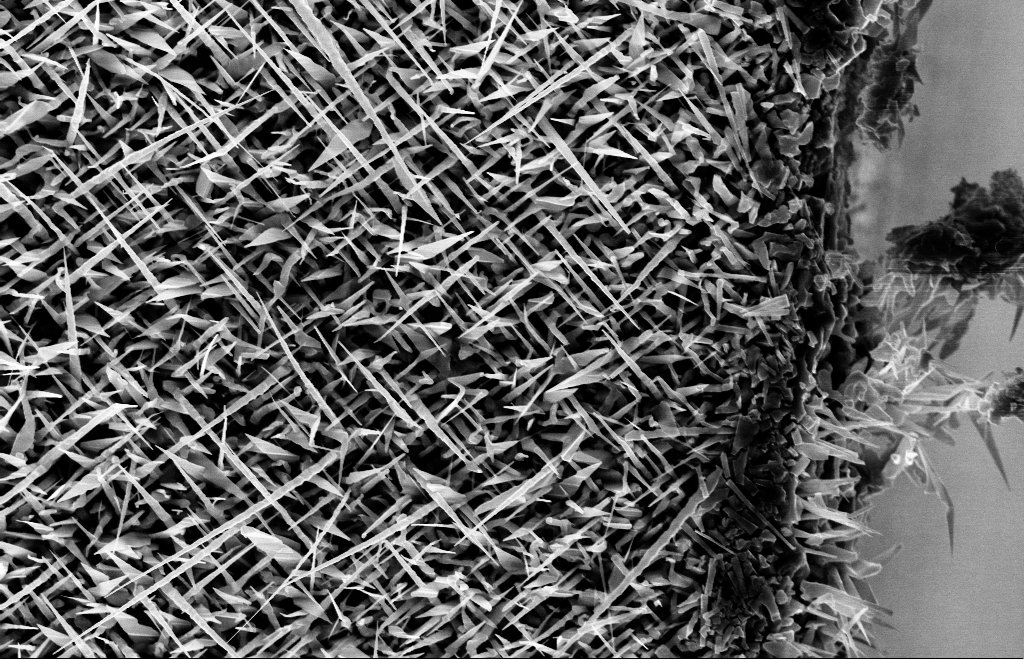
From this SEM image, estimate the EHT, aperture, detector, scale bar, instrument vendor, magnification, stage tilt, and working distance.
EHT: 10 kV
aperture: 30 µm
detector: InLens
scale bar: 1000 nm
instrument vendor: Zeiss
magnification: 20 K X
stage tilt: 0°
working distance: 8 mm